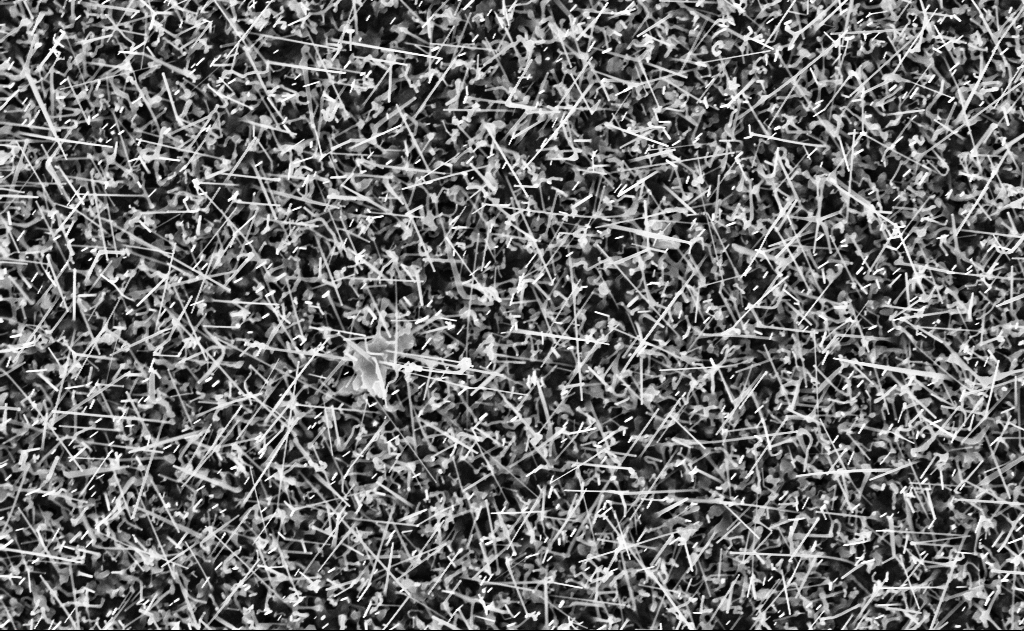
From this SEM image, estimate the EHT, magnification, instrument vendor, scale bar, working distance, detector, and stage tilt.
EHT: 10 kV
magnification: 20 K X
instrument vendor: Zeiss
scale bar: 2000 nm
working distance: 10 mm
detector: InLens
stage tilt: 0°